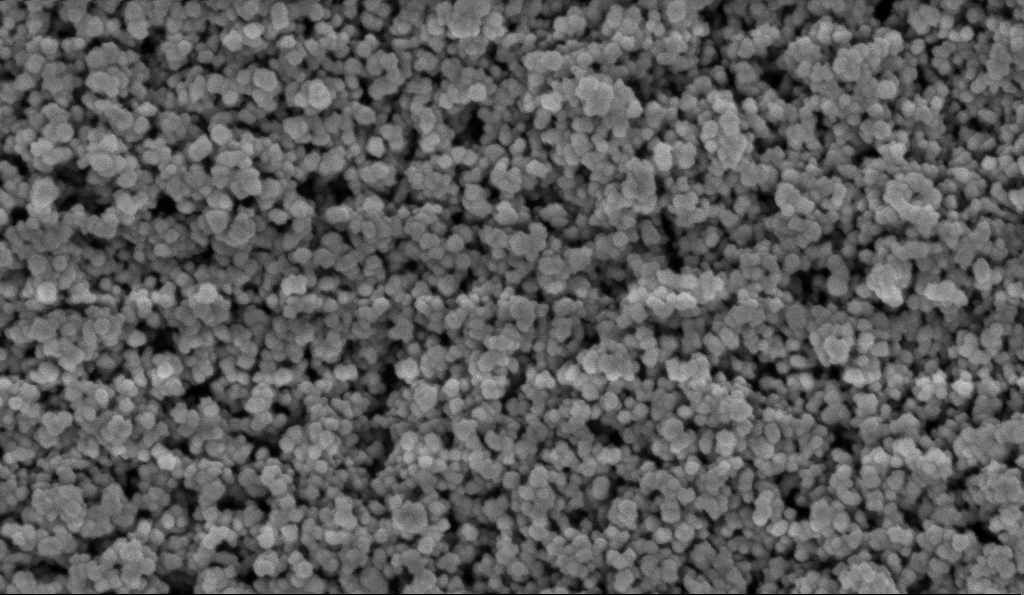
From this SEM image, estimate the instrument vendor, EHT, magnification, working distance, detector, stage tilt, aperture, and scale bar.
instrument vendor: Zeiss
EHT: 5 kV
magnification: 135 K X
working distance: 5.9 mm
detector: InLens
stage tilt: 0°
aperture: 30 µm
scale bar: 200 nm